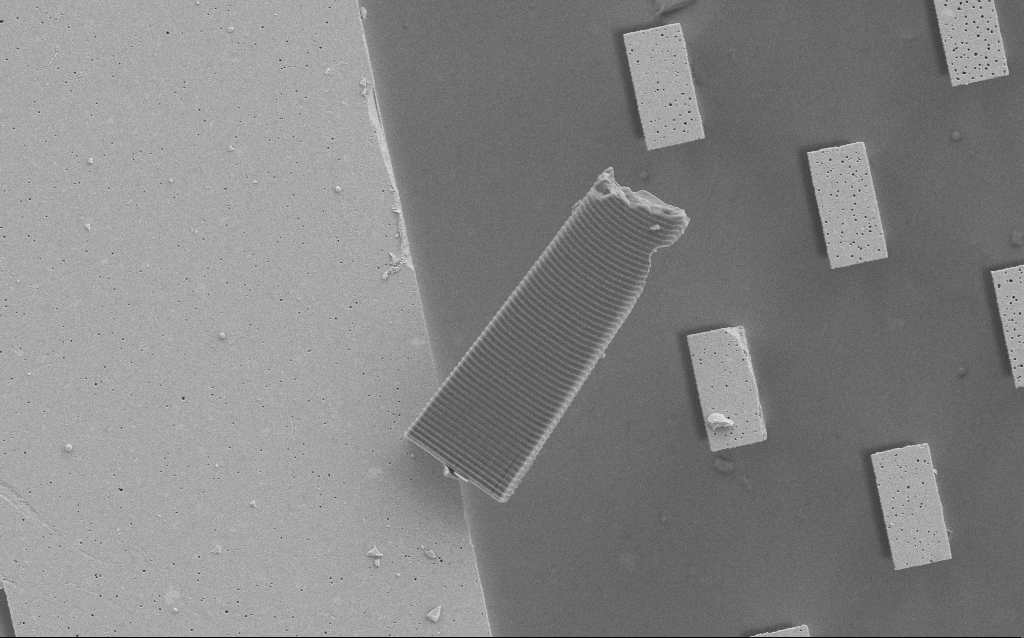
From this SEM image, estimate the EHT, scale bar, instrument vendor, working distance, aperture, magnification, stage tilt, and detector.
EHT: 5 kV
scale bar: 10000 nm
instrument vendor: Zeiss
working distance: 8 mm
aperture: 30 µm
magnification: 5.67 K X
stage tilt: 0°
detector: SE2